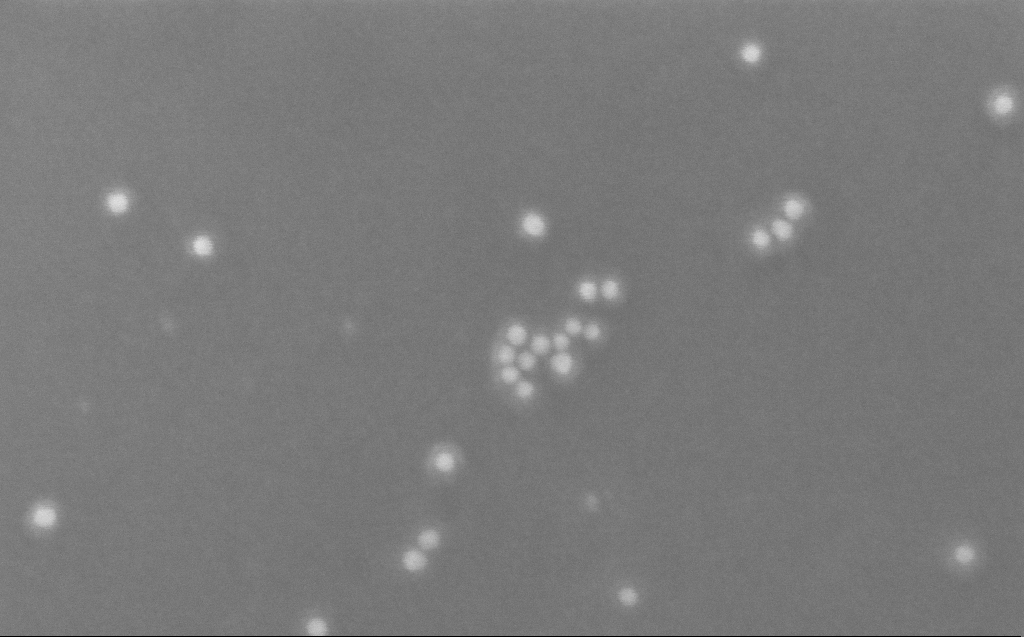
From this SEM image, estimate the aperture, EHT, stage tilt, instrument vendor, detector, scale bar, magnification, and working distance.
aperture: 30 µm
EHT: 10 kV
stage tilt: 0°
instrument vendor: Zeiss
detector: InLens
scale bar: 100 nm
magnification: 444.07 K X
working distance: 3 mm